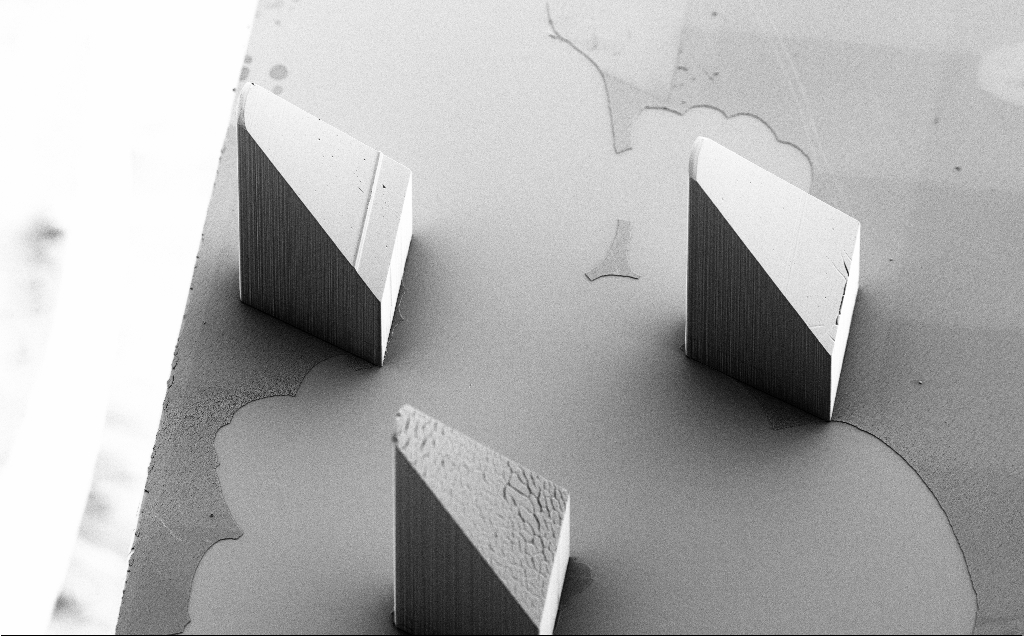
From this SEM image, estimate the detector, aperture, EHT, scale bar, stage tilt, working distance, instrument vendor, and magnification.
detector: SE2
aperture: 30 µm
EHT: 5 kV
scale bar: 100000 nm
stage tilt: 40°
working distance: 9 mm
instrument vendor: Zeiss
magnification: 0.168 K X